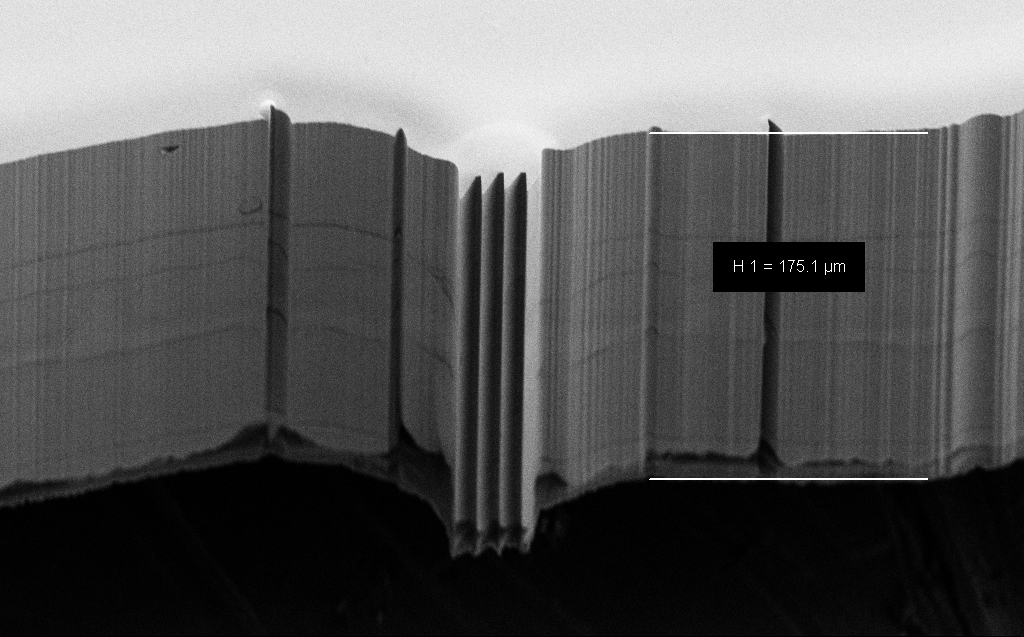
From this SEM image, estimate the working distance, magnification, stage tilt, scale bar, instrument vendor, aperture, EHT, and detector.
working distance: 4 mm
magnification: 0.726 K X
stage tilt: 45°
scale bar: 20000 nm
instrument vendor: Zeiss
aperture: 30 µm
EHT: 10 kV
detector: SE2